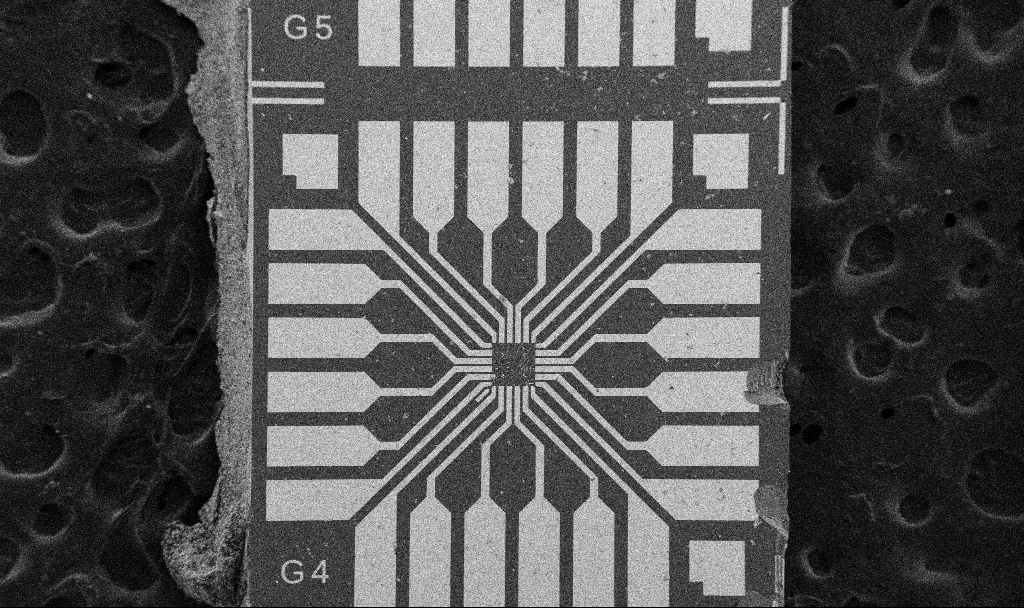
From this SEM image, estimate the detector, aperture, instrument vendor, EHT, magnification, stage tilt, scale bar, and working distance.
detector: SE2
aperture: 30 µm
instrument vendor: Zeiss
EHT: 5 kV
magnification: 0.1 K X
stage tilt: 0°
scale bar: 200000 nm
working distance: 10.7 mm